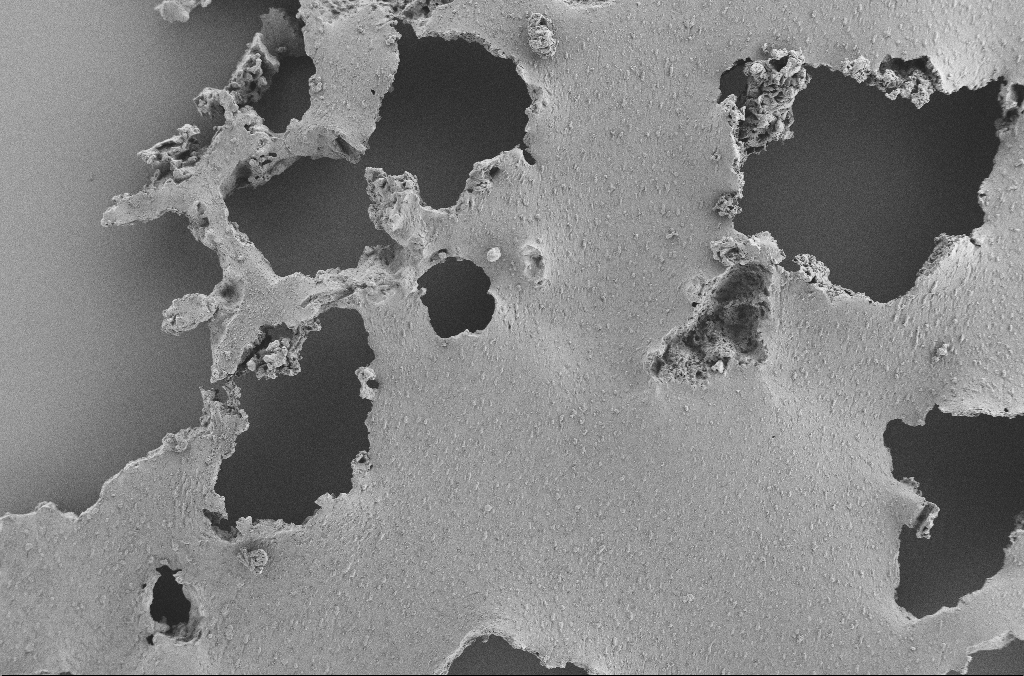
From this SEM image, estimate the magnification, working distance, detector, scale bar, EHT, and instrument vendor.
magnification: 0.25 K X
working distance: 3.6 mm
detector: SE2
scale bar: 100000 nm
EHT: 2 kV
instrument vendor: Zeiss